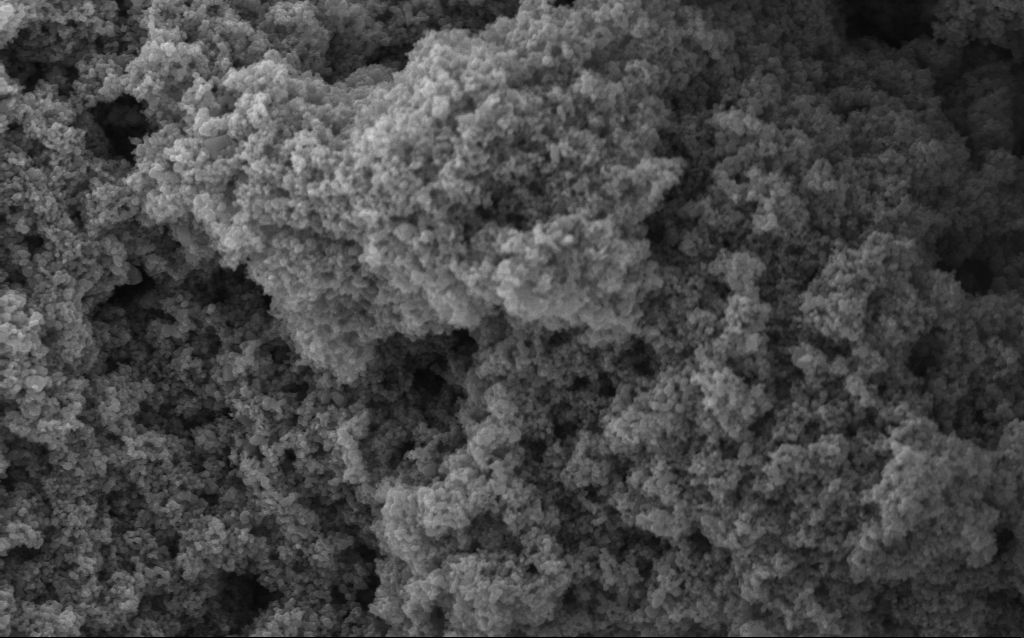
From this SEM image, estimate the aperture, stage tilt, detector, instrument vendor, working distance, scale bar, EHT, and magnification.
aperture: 30 µm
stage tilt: -0°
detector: InLens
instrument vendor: Zeiss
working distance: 4.2 mm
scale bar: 1000 nm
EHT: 5 kV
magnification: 68.59 K X